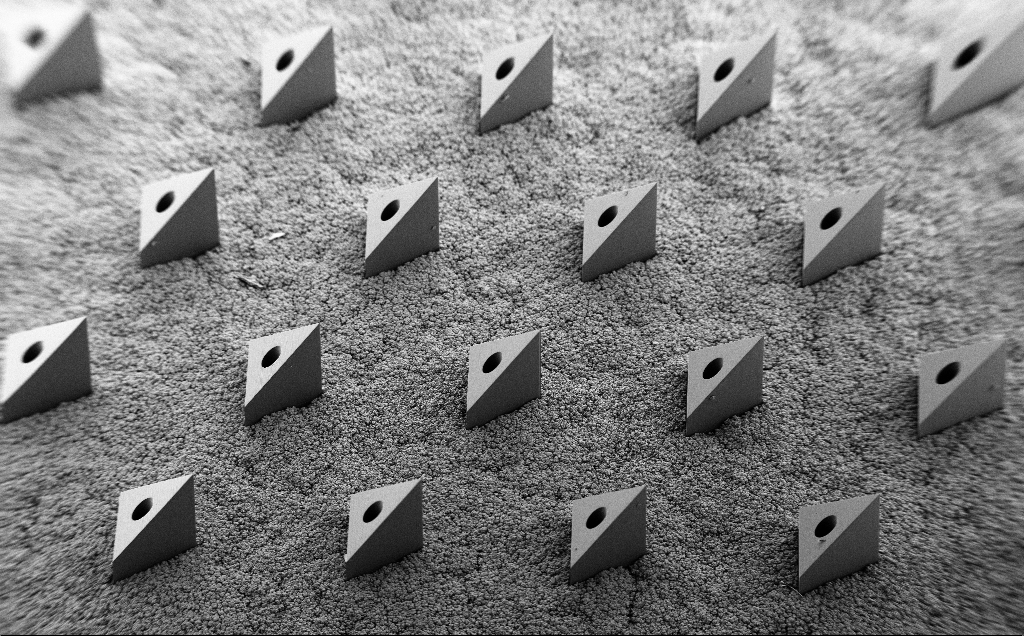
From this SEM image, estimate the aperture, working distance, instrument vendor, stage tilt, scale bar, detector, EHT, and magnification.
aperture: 30 µm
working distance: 9 mm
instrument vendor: Zeiss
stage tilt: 35°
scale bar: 200000 nm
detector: SE2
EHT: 5 kV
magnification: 0.082 K X